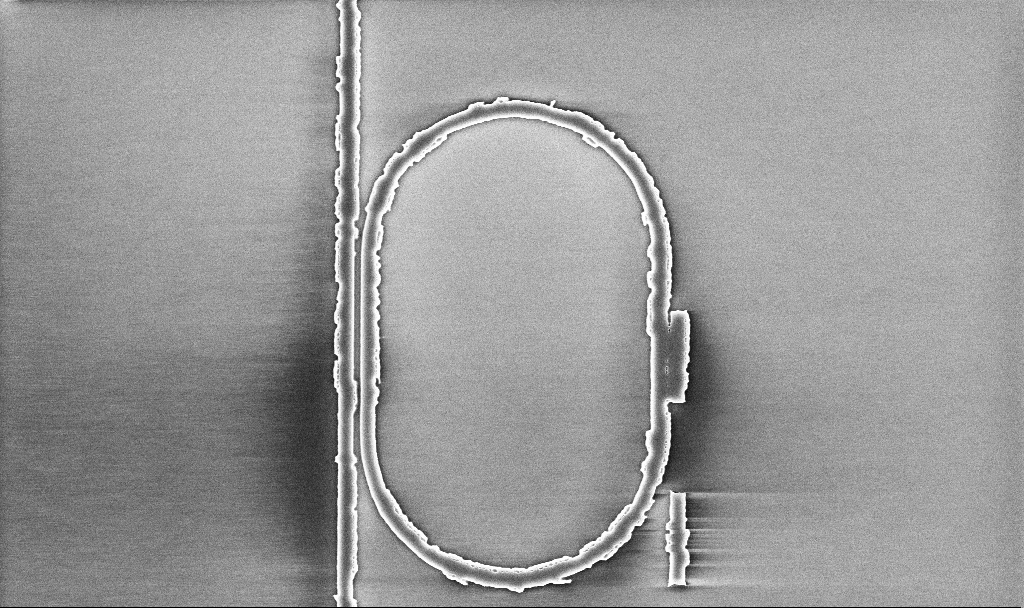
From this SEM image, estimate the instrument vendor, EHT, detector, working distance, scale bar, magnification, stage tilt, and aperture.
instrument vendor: Zeiss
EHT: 5 kV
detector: InLens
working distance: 10.1 mm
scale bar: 2000 nm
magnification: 11.31 K X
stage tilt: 0°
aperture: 30 µm